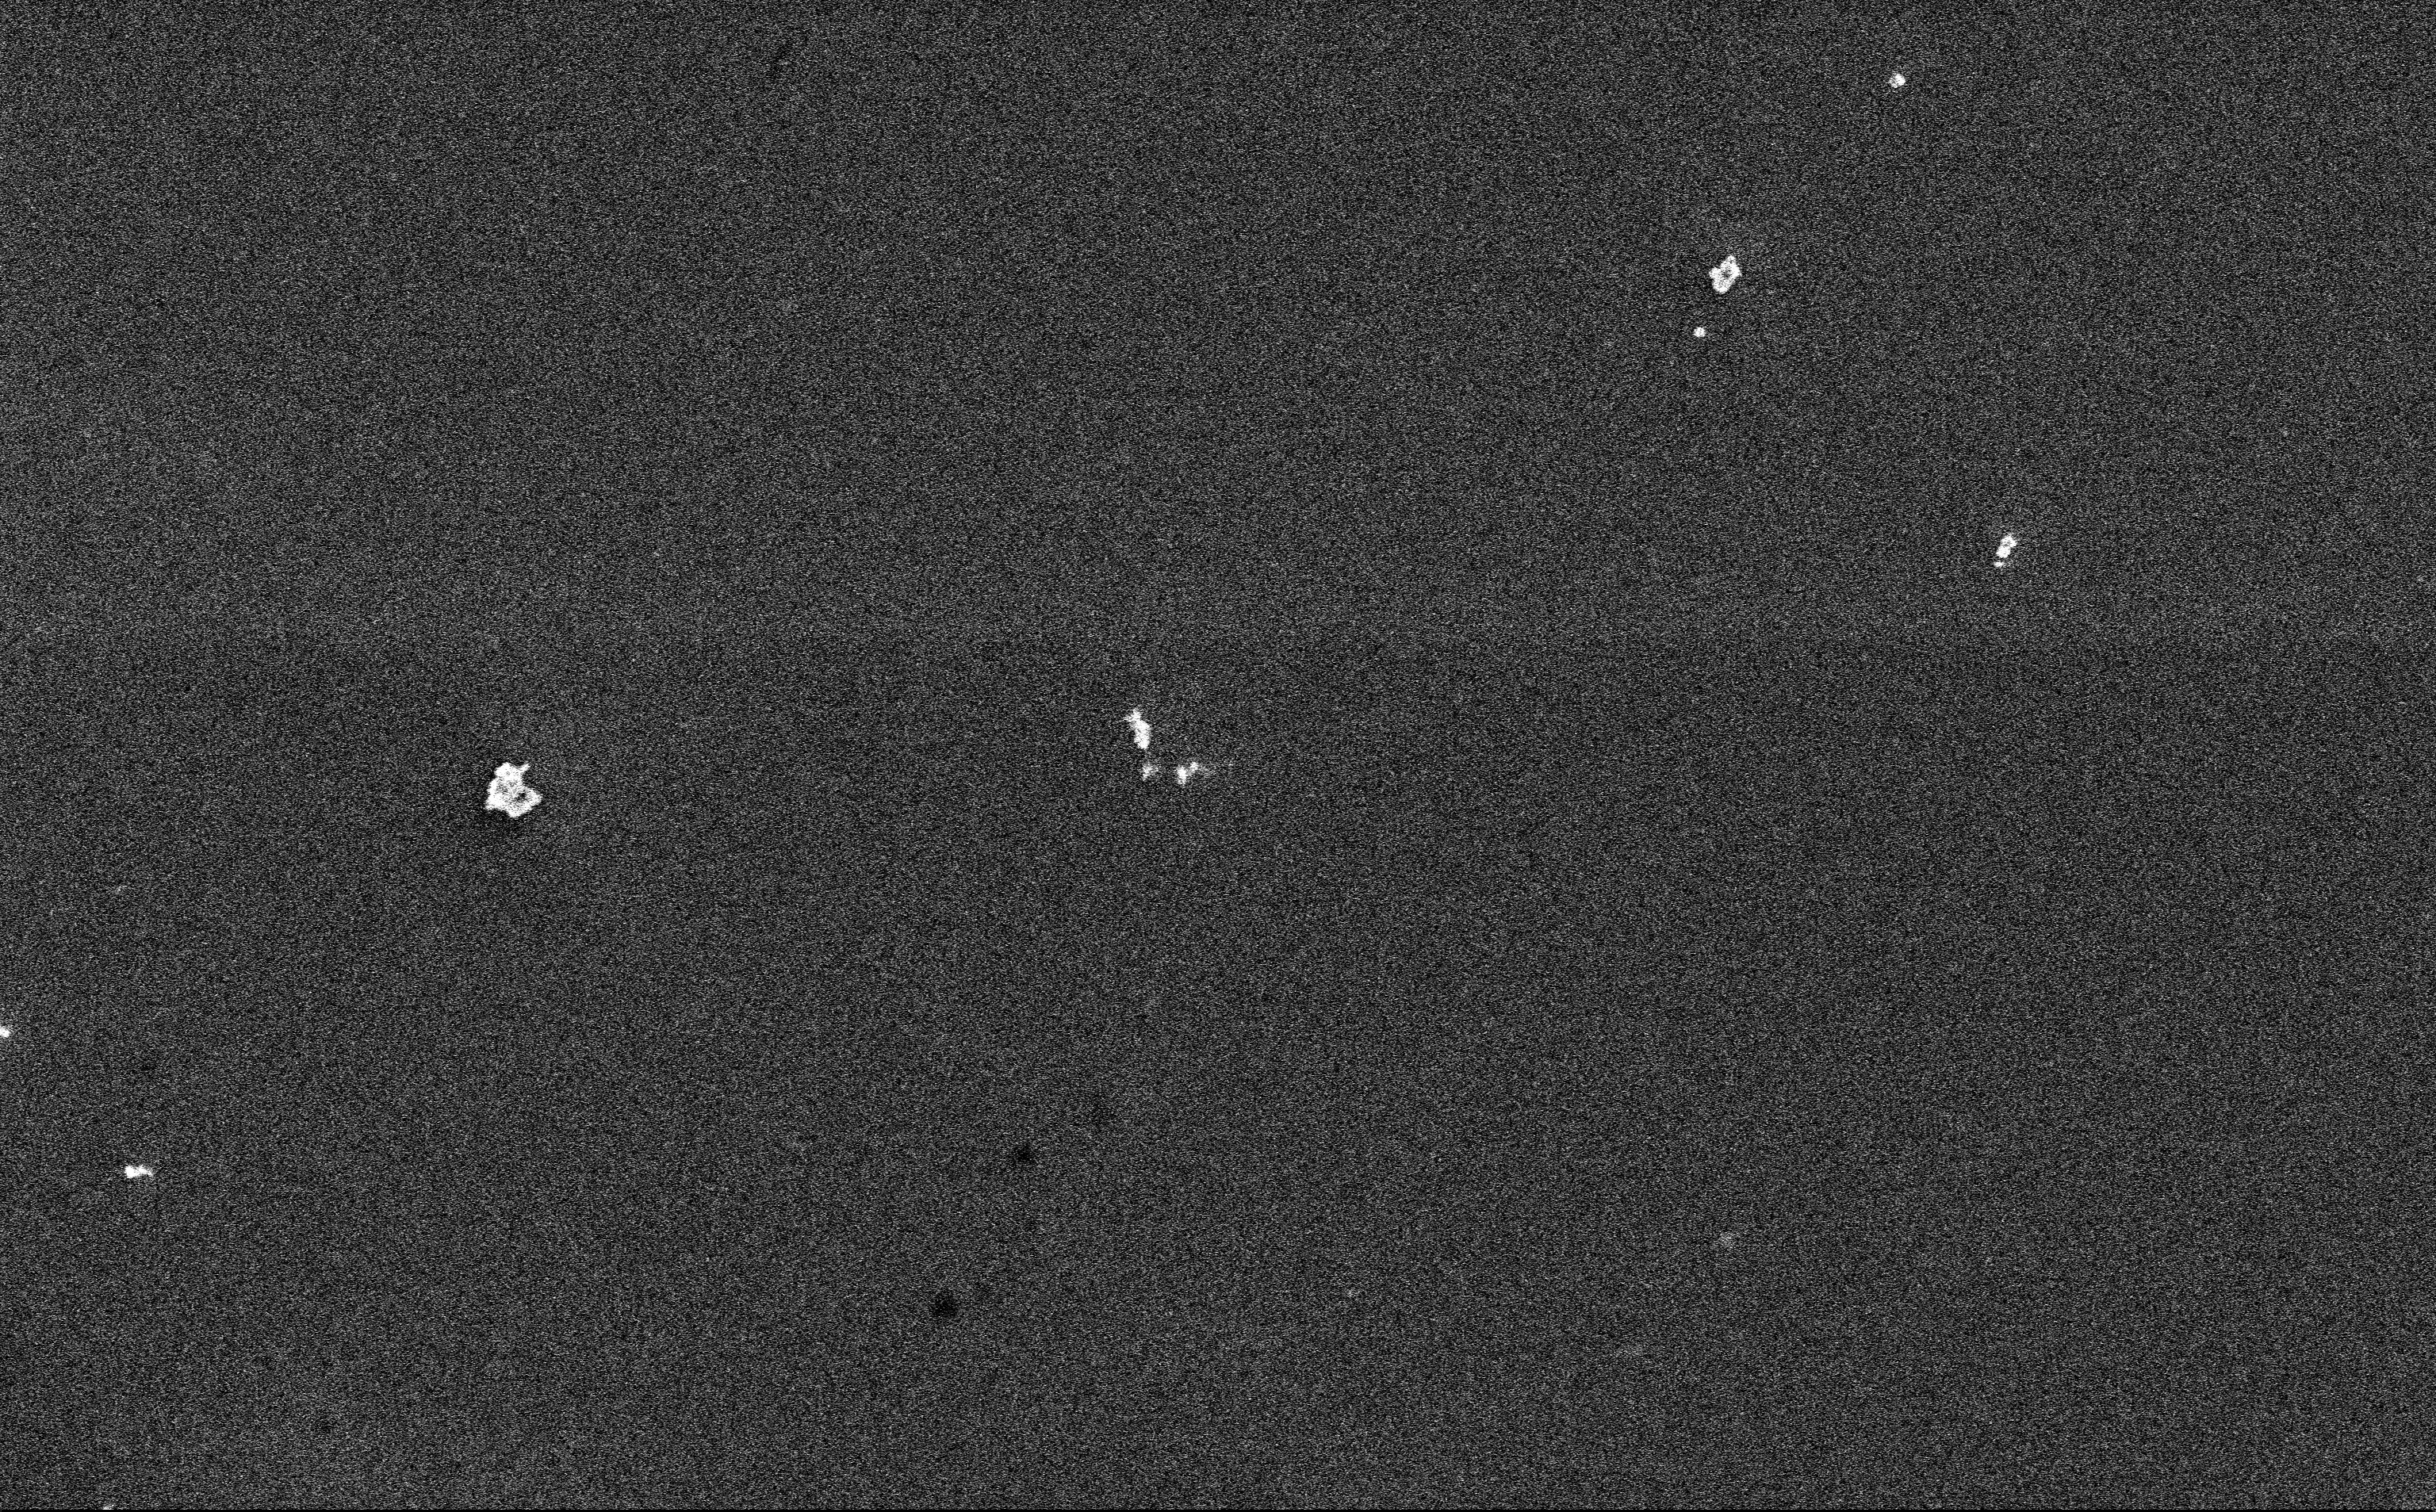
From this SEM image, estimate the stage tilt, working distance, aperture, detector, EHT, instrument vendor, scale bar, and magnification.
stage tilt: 0°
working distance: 3 mm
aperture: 30 µm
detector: InLens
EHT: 3 kV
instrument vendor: Zeiss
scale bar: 2000 nm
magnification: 12.85 K X